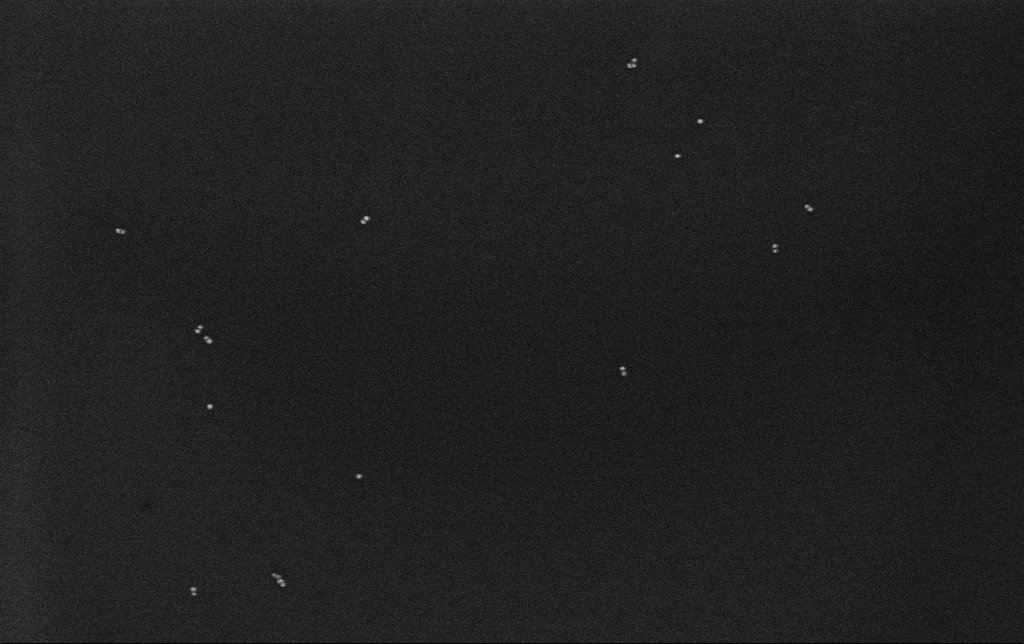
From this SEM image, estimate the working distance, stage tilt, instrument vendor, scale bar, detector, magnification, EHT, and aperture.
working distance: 3.2 mm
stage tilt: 0°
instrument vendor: Zeiss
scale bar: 200 nm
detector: InLens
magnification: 100 K X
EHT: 10 kV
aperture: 30 µm